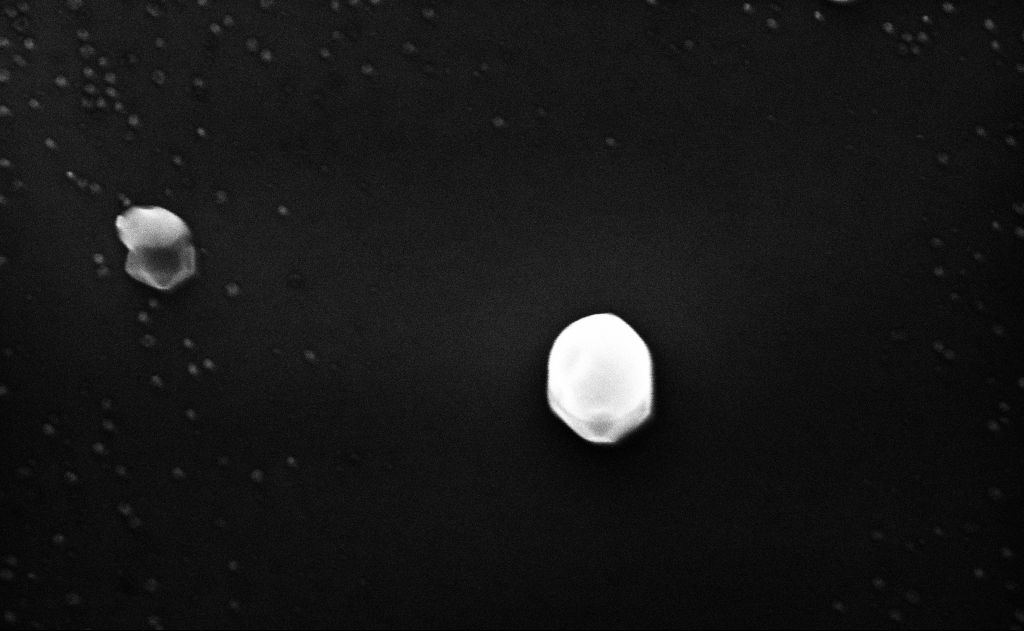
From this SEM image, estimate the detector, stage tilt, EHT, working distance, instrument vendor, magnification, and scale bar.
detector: InLens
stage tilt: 0°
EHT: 10 kV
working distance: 10 mm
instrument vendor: Zeiss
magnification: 100 K X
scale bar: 200 nm